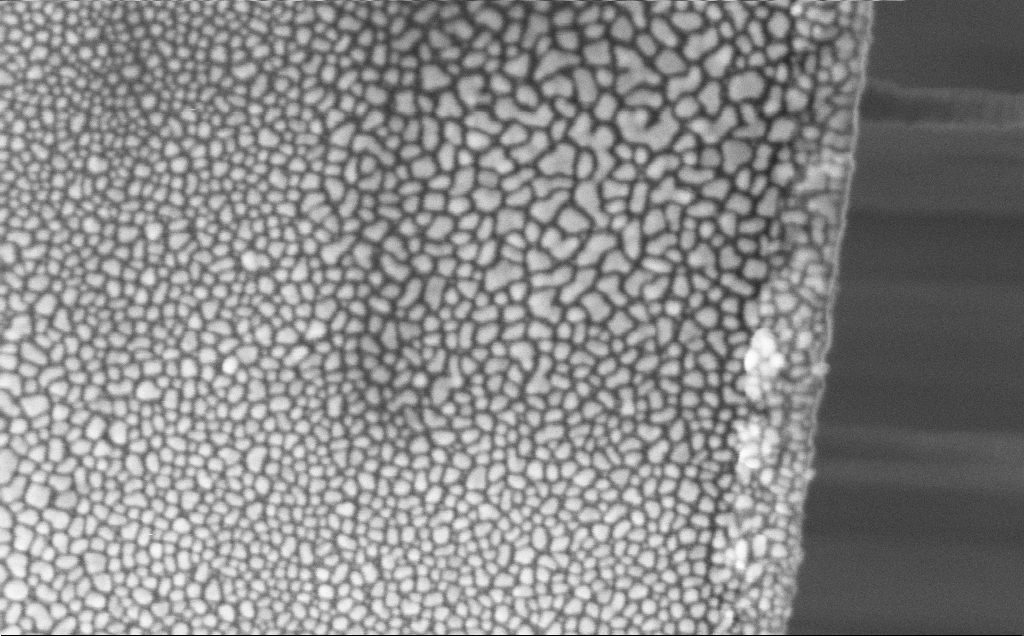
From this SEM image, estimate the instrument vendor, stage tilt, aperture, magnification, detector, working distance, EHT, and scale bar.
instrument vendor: Zeiss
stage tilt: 0°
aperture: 30 µm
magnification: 109.89 K X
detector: InLens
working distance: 12 mm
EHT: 5 kV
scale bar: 200 nm